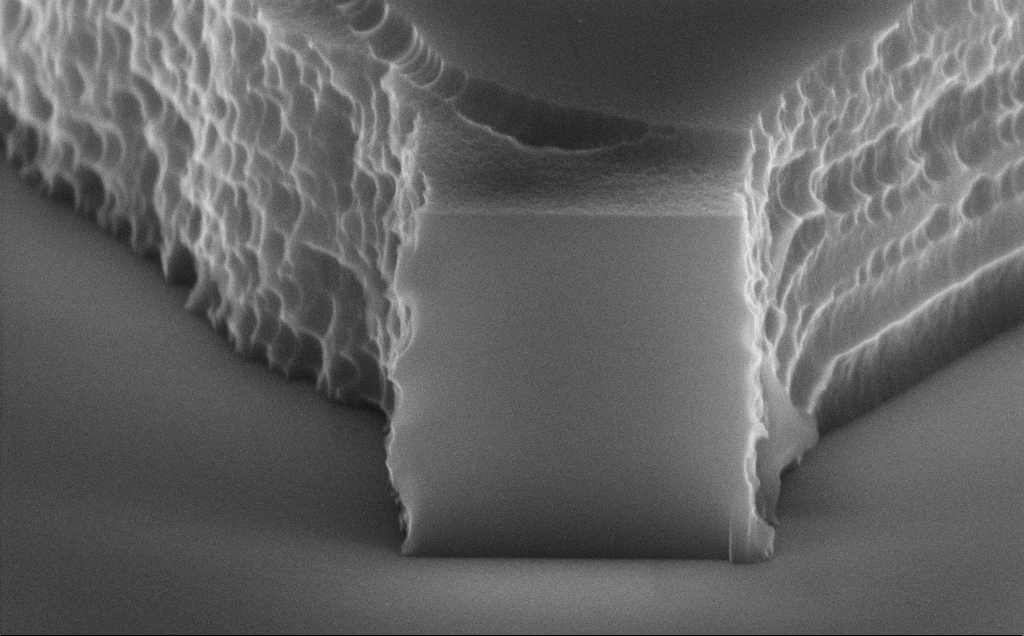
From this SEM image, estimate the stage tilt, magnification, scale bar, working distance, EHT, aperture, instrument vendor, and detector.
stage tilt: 70°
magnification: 59.96 K X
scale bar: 1000 nm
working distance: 10 mm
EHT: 10 kV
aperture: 30 µm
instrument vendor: Zeiss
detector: InLens